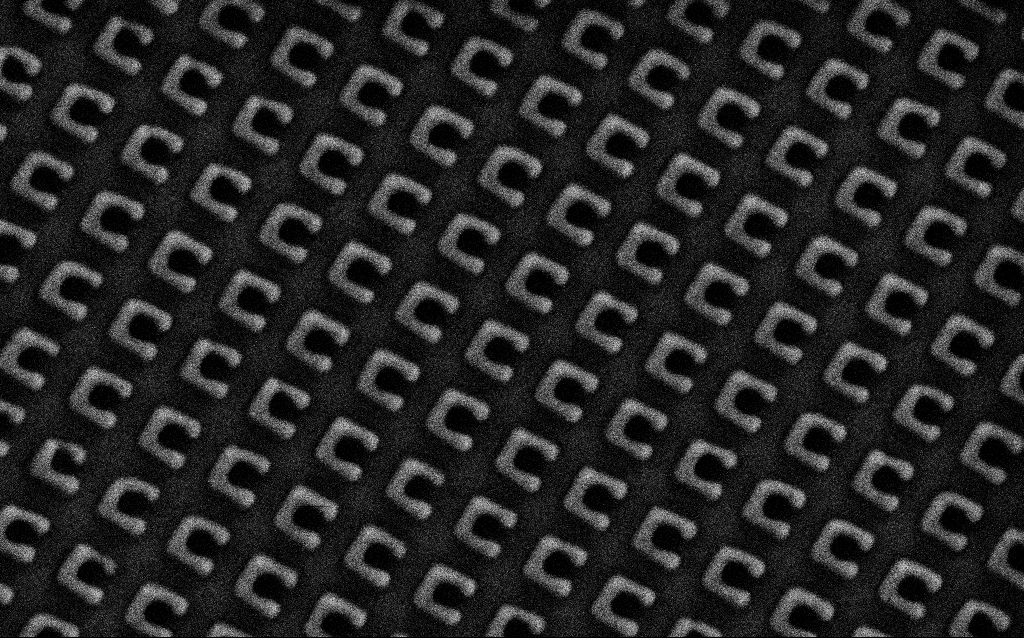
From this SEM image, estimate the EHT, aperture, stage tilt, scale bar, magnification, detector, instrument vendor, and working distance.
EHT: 1.5 kV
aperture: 30 µm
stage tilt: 0°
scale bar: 1000 nm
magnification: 62.87 K X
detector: SE2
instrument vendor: Zeiss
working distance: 8 mm